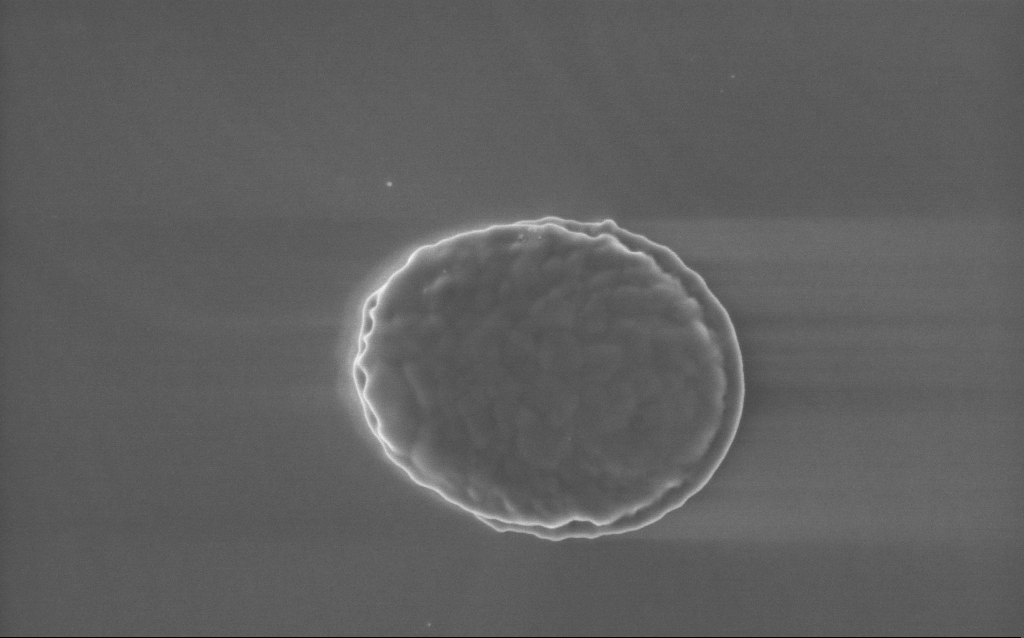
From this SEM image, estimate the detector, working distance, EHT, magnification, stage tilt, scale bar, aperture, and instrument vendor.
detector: InLens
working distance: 3 mm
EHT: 5 kV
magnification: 44 K X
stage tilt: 0°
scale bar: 1000 nm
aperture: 30 µm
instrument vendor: Zeiss